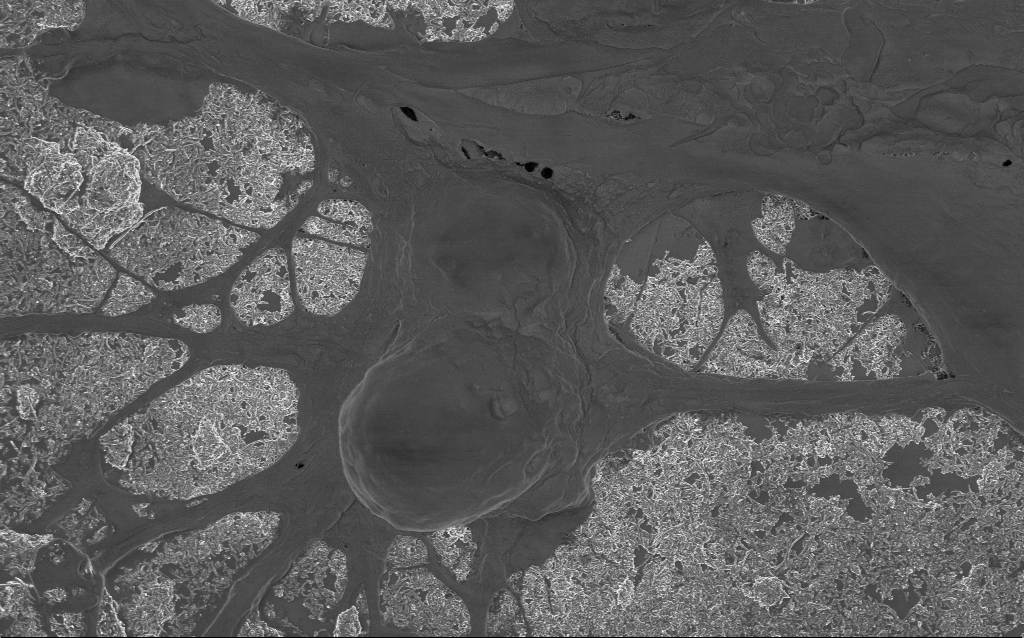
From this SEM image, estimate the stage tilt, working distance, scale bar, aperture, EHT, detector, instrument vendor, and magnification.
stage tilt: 0°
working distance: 6 mm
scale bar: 2000 nm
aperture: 30 µm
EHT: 1 kV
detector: InLens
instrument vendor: Zeiss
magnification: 10 K X